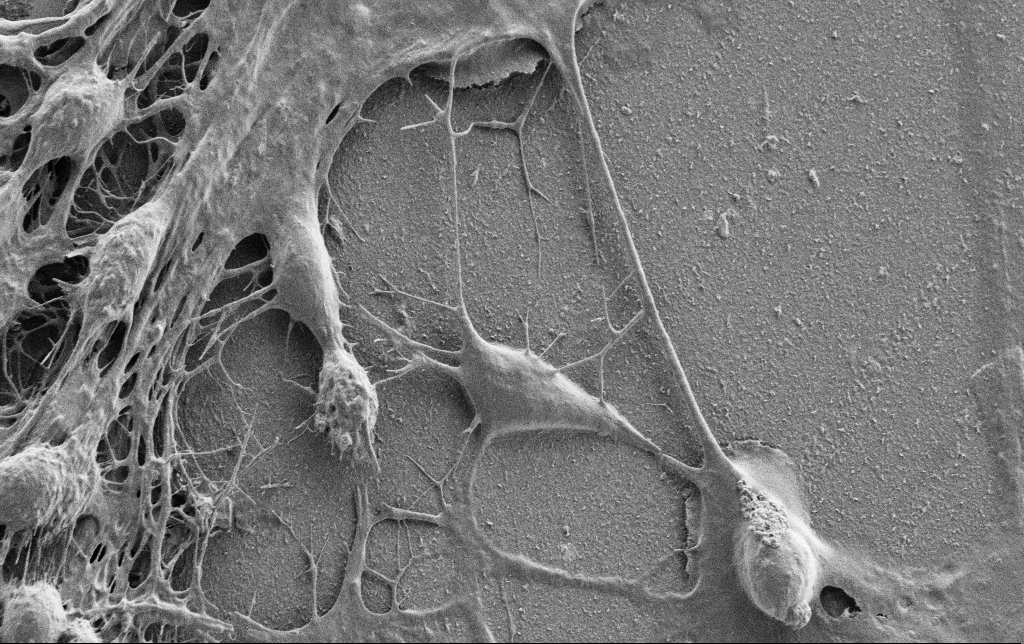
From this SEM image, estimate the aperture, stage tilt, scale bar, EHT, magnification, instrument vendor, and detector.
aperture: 30 µm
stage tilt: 0°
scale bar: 10000 nm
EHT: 0.9 kV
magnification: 5 K X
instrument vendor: Zeiss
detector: SE2